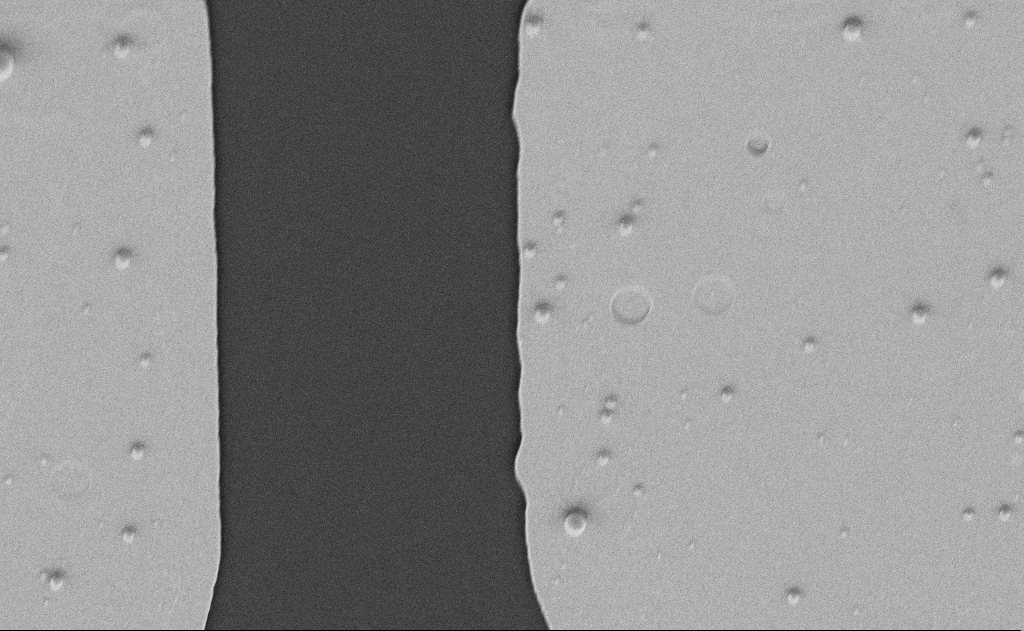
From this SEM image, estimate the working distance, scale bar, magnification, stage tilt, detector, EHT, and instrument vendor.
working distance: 6 mm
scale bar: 1000 nm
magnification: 12.5 K X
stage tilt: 30.1°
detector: SE2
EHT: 5 kV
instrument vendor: Zeiss